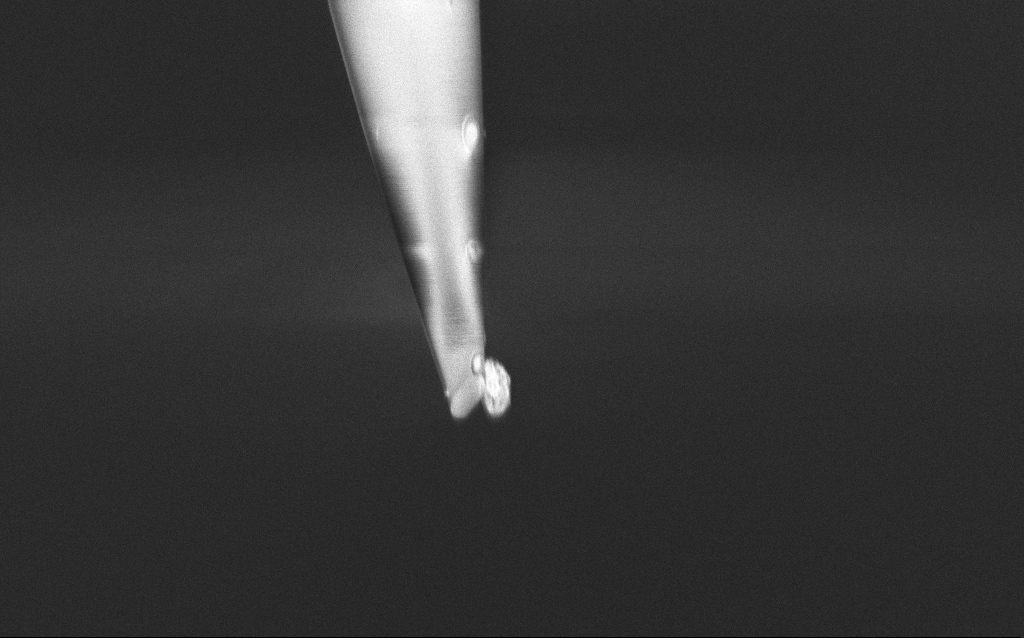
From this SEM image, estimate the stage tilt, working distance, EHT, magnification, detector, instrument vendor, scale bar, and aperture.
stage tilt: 45°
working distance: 5 mm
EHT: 1 kV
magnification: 19.71 K X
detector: InLens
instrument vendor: Zeiss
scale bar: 2000 nm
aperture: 30 µm